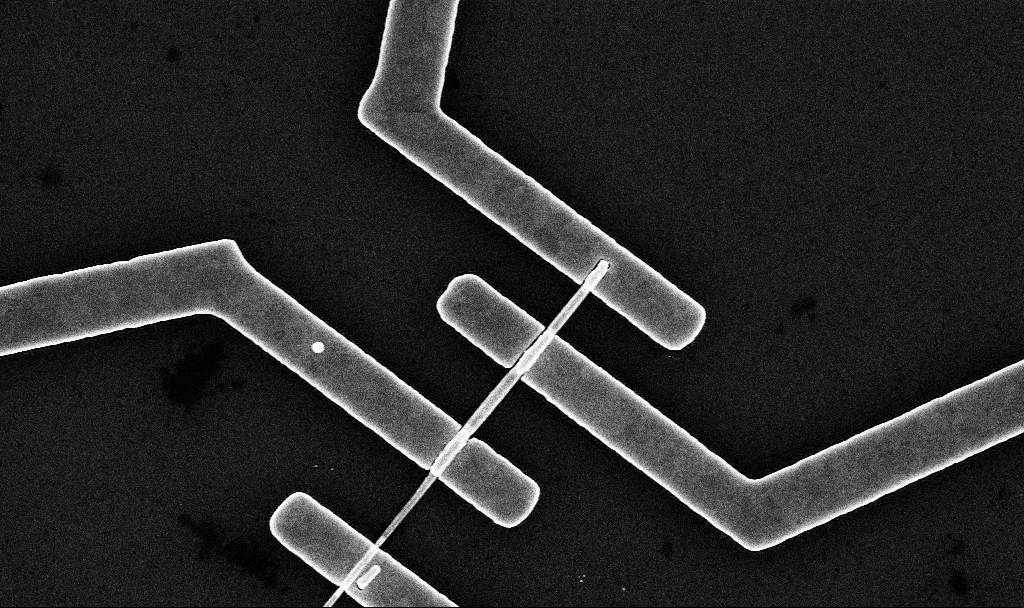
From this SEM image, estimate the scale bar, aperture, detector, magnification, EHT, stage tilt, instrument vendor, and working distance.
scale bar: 2000 nm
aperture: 30 µm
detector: InLens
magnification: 29 K X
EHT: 10 kV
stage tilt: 0°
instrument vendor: Zeiss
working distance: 7.6 mm